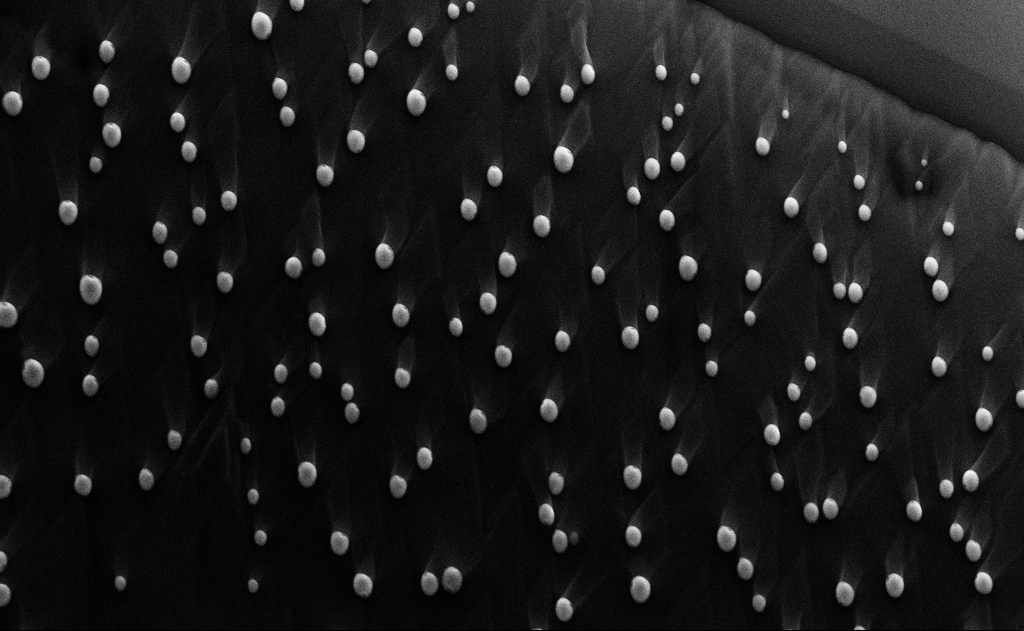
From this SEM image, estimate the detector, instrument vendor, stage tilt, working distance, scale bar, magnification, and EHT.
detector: InLens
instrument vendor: Zeiss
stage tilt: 0°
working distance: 12 mm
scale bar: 2000 nm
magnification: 10 K X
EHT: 10 kV